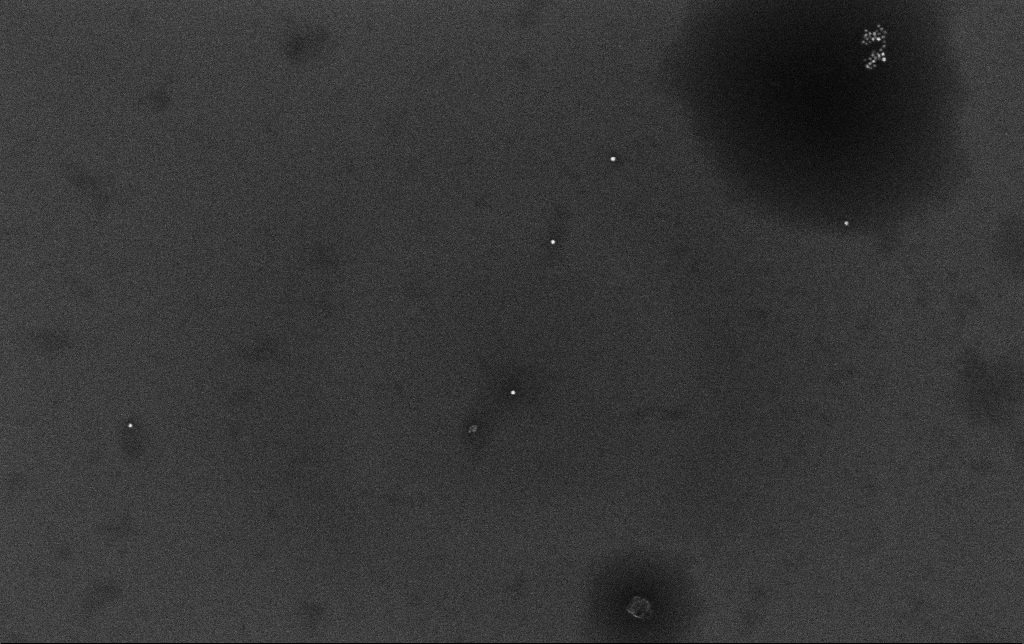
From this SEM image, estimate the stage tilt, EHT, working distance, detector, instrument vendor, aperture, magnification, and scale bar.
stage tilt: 0°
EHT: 10 kV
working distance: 3.4 mm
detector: InLens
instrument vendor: Zeiss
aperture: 30 µm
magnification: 100 K X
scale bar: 200 nm